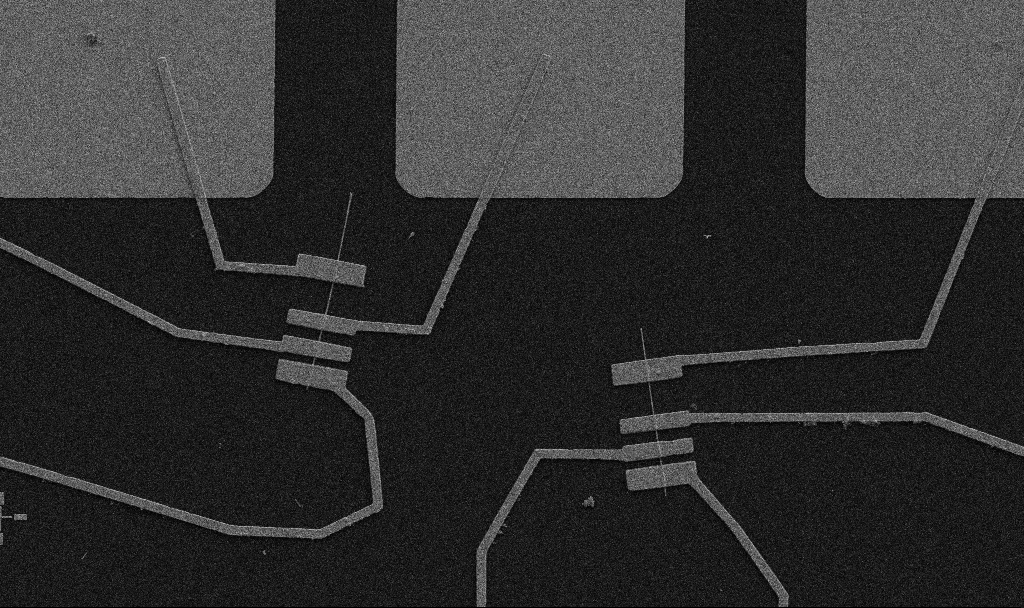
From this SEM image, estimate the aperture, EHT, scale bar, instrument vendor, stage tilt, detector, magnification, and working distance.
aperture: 30 µm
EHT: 5 kV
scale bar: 10000 nm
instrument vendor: Zeiss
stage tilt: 0°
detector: SE2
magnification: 5 K X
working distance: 10.7 mm